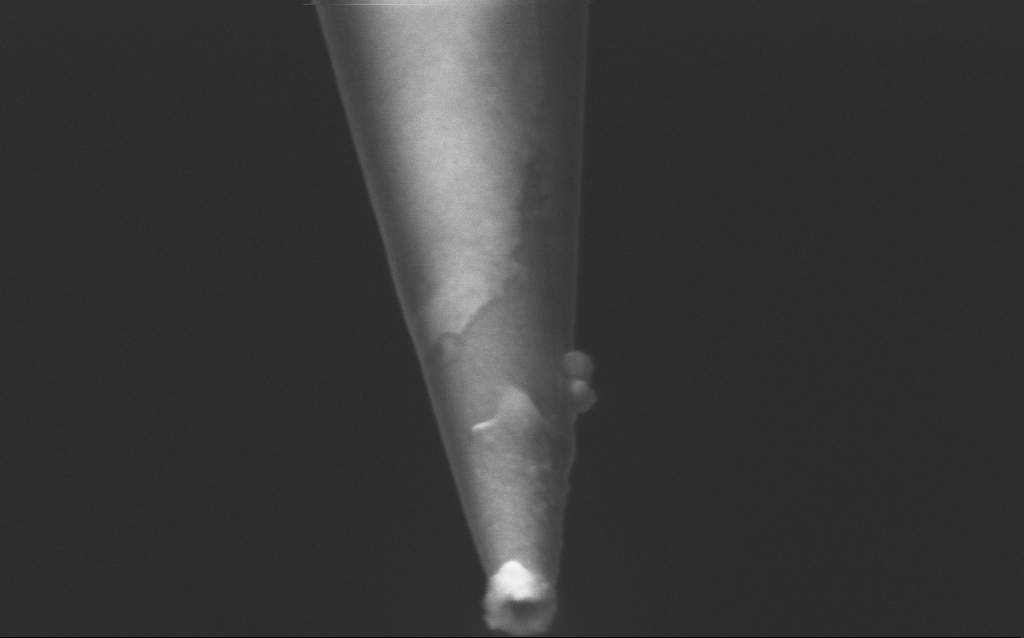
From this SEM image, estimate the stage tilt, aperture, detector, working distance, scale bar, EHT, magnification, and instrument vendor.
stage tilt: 45°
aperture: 30 µm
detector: InLens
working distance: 5 mm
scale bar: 200 nm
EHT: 2.5 kV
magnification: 250 K X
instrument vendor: Zeiss